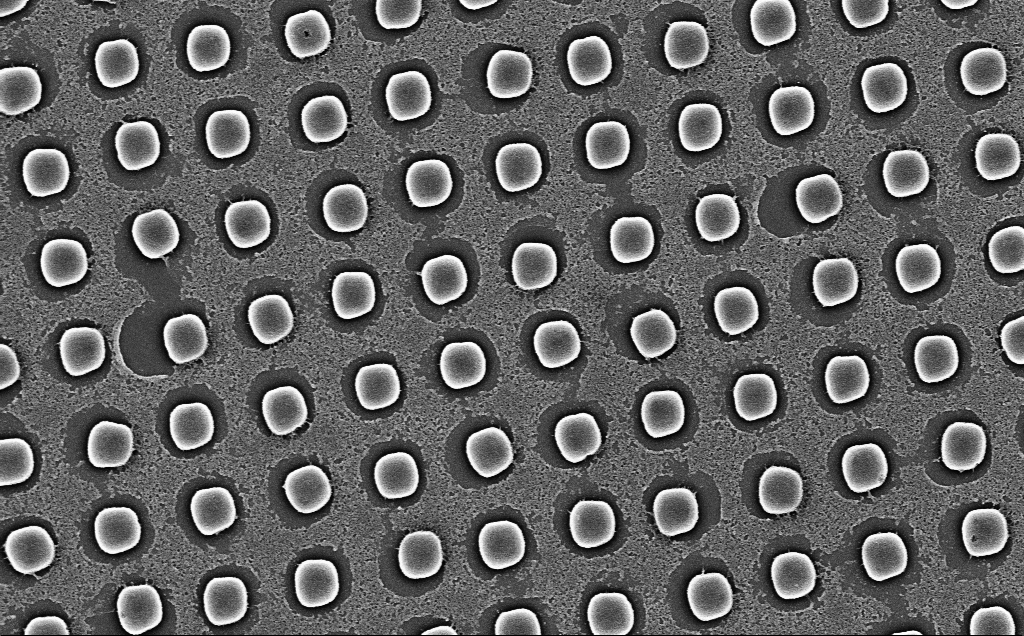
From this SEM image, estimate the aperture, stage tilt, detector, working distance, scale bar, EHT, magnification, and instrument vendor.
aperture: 30 µm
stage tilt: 0°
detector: InLens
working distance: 7 mm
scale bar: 2000 nm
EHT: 5 kV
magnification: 22.78 K X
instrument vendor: Zeiss